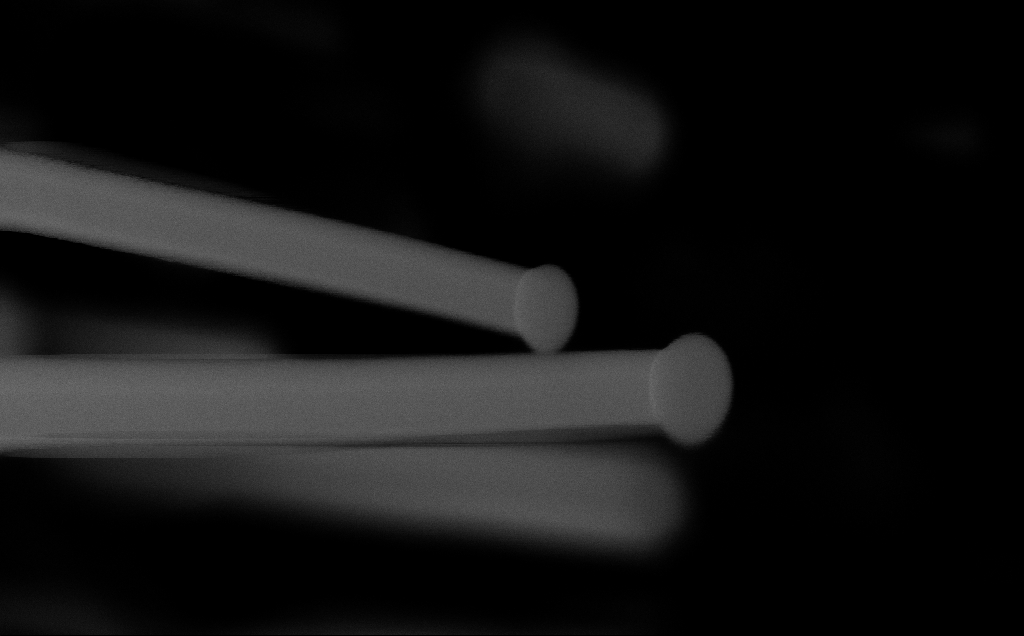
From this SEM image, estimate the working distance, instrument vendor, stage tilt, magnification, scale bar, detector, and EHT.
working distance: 6 mm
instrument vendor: Zeiss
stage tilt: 0°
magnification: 289.71 K X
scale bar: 100 nm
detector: InLens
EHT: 10 kV